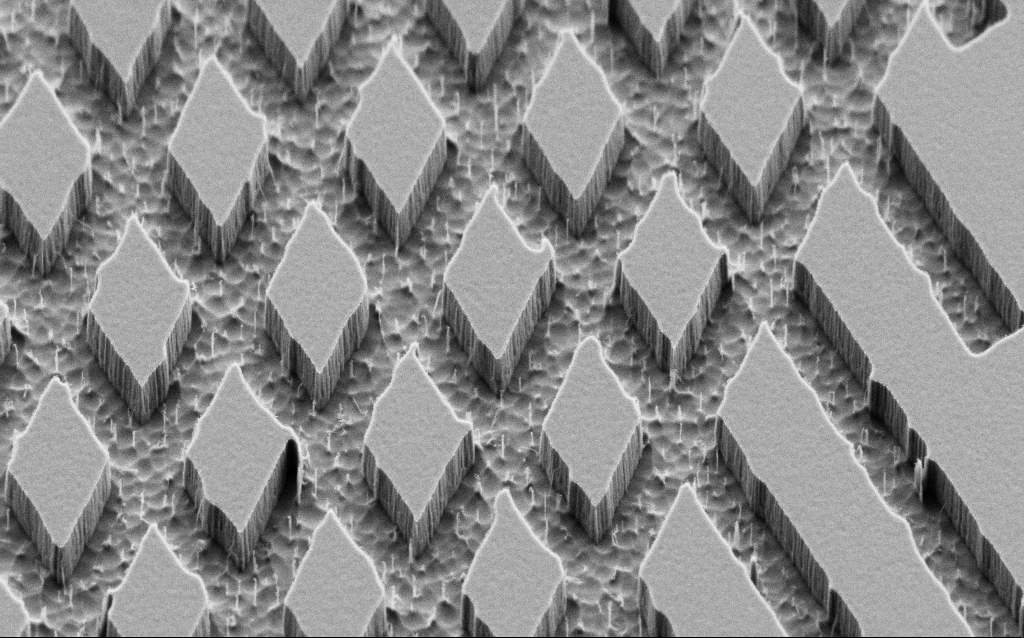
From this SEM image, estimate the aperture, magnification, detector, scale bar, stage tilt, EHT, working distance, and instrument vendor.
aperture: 30 µm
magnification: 22.72 K X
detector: SE2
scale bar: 1000 nm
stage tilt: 45°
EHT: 2 kV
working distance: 6 mm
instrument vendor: Zeiss